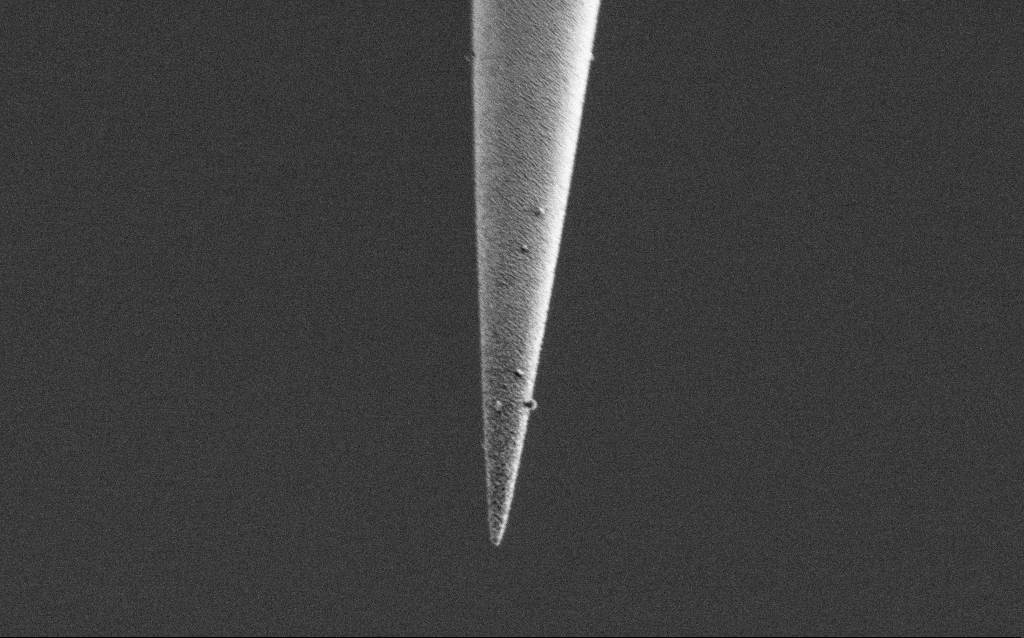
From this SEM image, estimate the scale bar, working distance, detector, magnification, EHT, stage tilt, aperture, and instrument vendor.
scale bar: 2000 nm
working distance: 6.7 mm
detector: SE2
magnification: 25 K X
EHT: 1 kV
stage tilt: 45°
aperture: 30 µm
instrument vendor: Zeiss